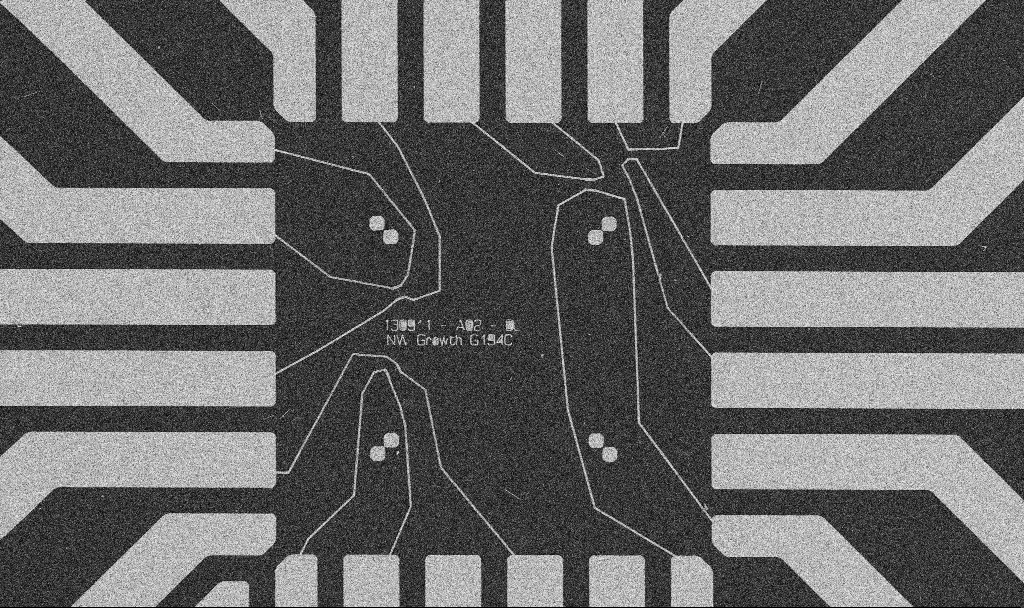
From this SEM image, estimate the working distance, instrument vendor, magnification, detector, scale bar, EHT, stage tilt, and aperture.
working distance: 10.6 mm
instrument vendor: Zeiss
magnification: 1 K X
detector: SE2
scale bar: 20000 nm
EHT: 5 kV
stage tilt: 0°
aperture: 30 µm